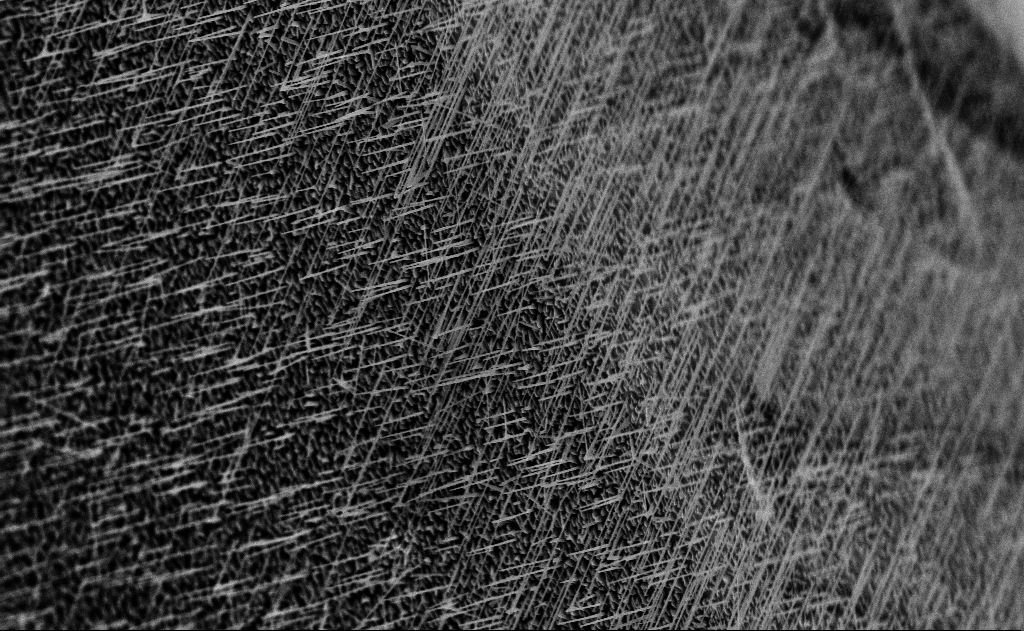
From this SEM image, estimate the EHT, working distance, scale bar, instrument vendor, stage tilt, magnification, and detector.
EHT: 10 kV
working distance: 14 mm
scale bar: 2000 nm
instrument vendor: Zeiss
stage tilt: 0°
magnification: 10 K X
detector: InLens